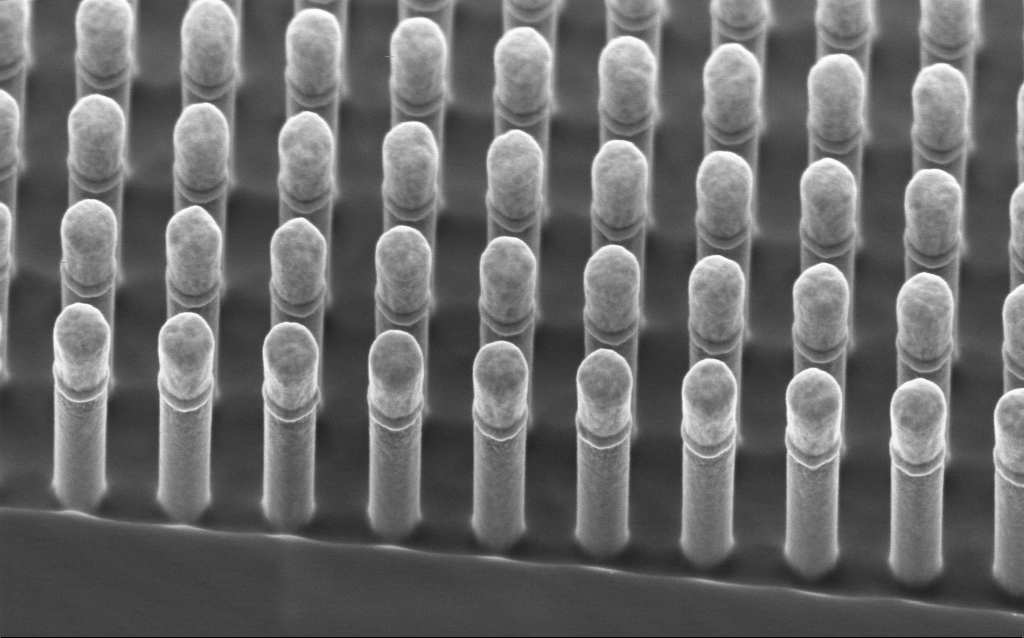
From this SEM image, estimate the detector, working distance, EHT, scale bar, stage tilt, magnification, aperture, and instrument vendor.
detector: InLens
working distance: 2.4 mm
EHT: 2 kV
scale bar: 200 nm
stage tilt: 45°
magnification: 77.4 K X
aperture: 30 µm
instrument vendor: Zeiss